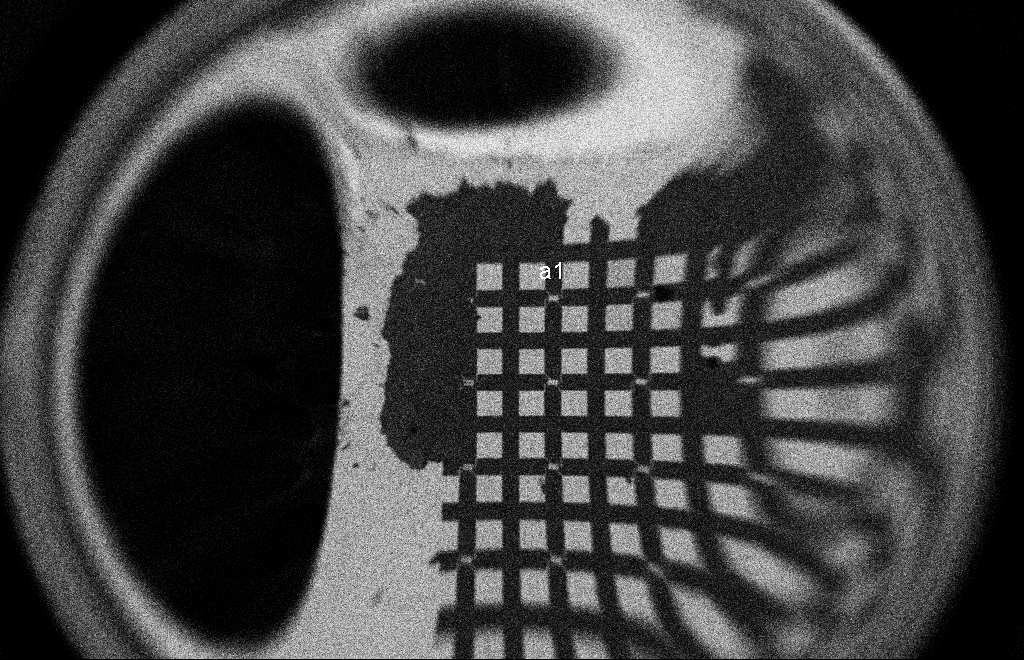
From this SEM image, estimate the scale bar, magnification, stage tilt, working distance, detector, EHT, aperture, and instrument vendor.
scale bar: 200000 nm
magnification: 0.061 K X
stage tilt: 0°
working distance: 9 mm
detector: SE2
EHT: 2 kV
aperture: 20 µm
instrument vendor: Zeiss